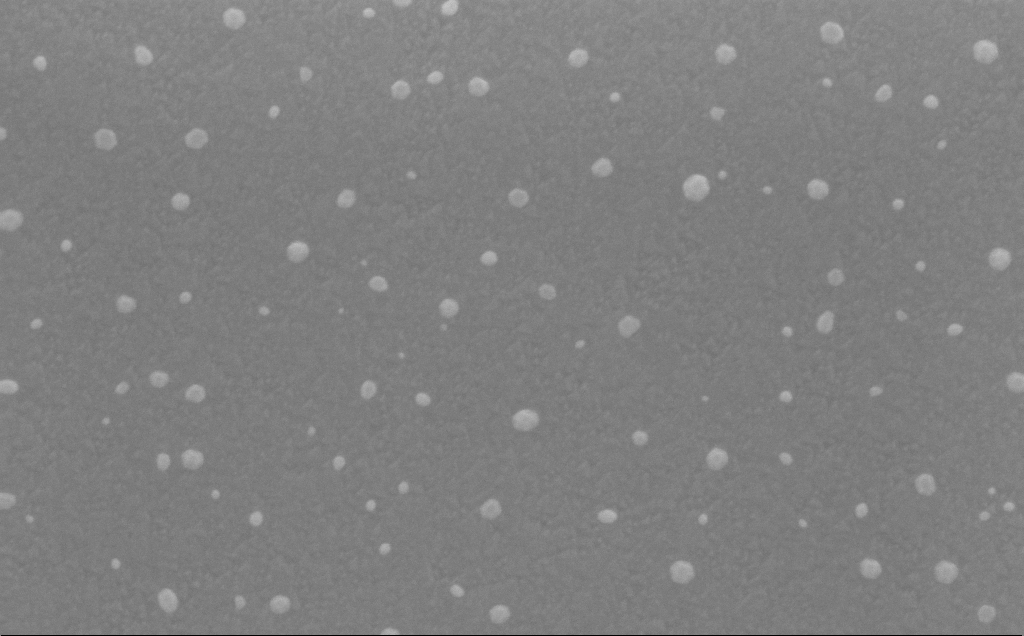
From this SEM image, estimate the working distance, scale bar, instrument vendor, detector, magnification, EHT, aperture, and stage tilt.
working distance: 5 mm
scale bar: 100 nm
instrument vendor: Zeiss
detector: InLens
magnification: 143.2 K X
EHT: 10 kV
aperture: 30 µm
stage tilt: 15.5°